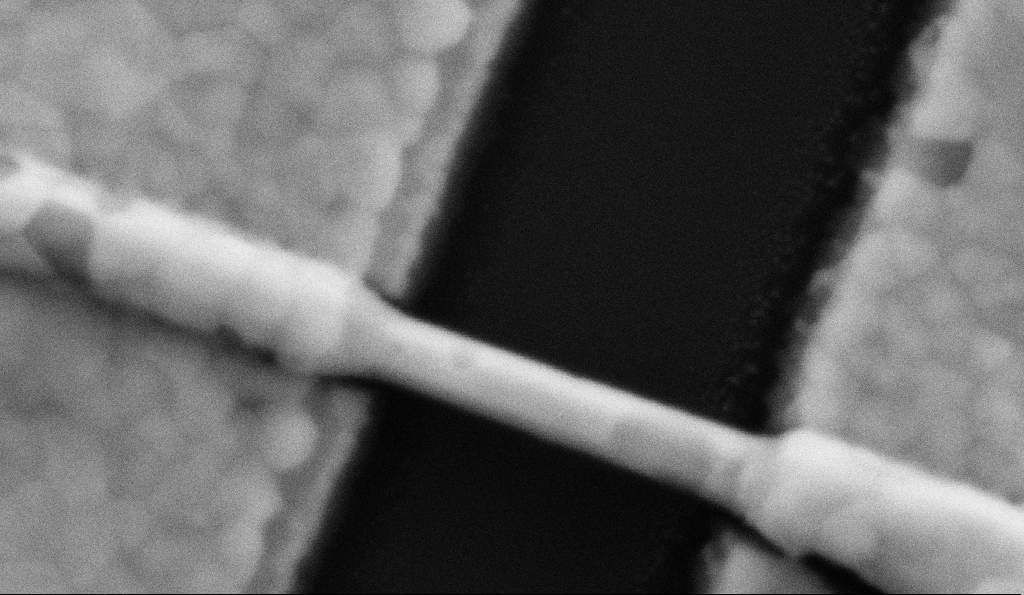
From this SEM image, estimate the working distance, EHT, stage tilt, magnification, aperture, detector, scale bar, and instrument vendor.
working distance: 8.5 mm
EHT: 5 kV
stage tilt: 0°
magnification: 300 K X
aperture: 30 µm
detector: SE2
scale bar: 200 nm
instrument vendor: Zeiss